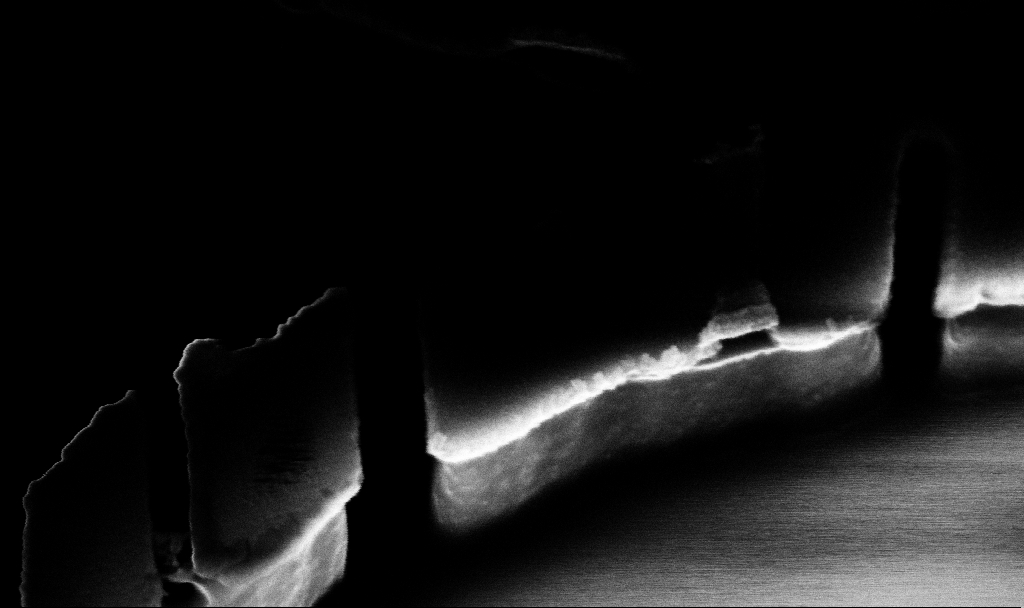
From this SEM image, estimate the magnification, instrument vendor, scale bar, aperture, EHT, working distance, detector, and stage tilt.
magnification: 171.69 K X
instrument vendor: Zeiss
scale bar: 200 nm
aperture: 30 µm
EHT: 5 kV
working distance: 8.8 mm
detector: InLens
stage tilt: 45°